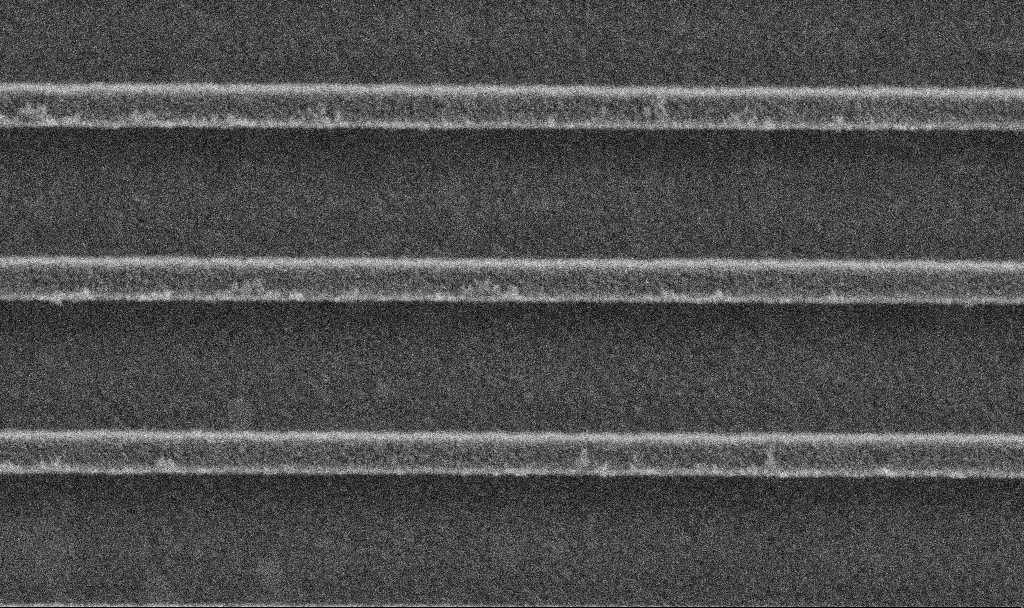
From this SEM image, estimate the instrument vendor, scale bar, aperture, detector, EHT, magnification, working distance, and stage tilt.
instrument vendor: Zeiss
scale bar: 200 nm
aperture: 30 µm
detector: SE2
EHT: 5 kV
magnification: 80.09 K X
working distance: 2.9 mm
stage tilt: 0°